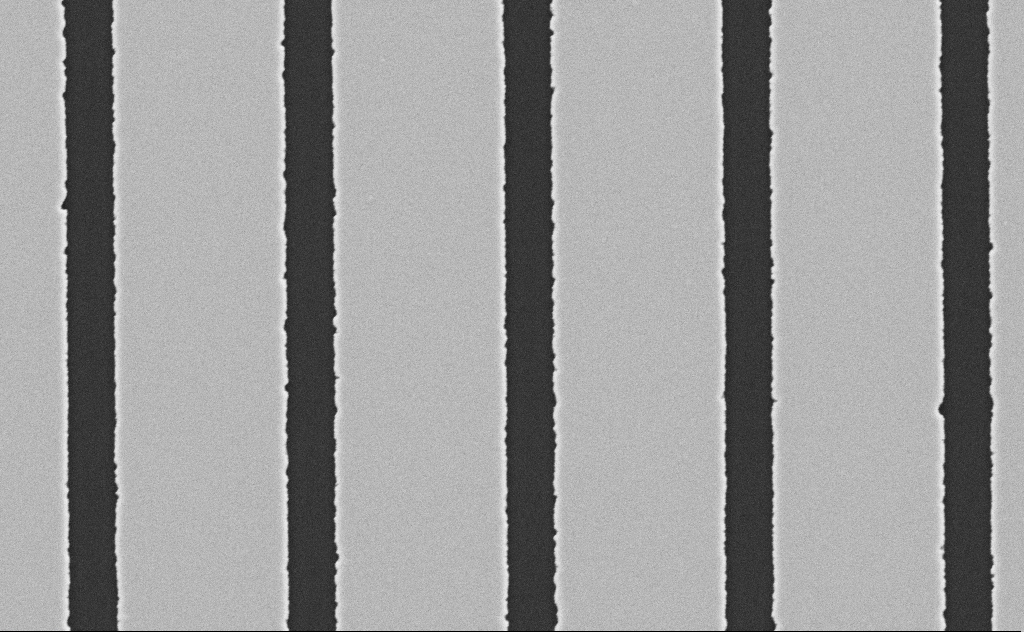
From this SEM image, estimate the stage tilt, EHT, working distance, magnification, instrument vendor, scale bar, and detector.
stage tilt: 0°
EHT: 5 kV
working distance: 8 mm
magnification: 20.1 K X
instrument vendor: Zeiss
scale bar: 2000 nm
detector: SE2